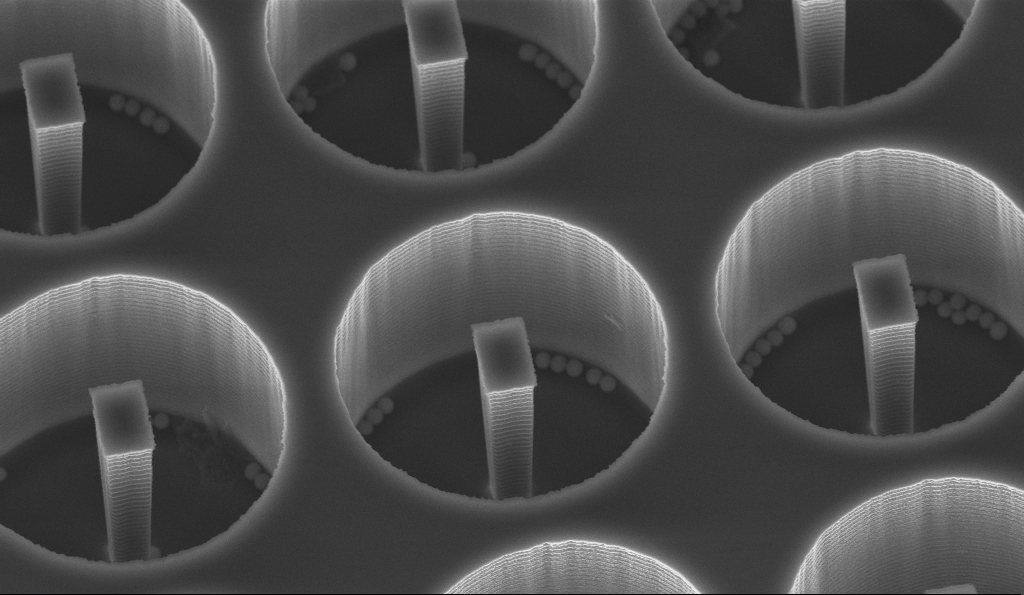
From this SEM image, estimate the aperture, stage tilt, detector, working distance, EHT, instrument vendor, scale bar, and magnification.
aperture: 30 µm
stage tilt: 30°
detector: InLens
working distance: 14.1 mm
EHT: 10 kV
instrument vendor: Zeiss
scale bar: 2000 nm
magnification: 10 K X